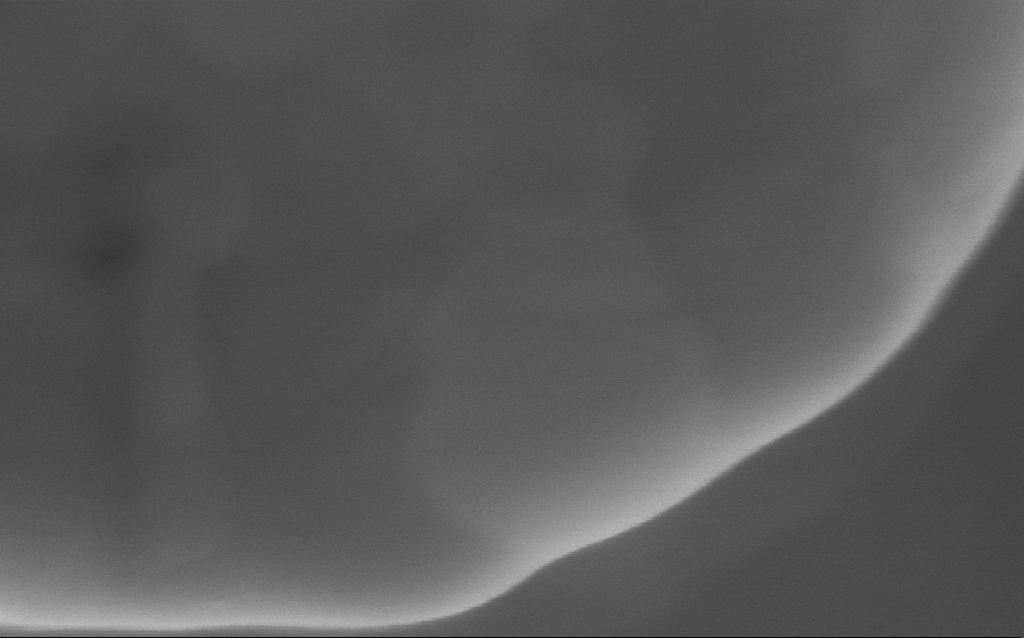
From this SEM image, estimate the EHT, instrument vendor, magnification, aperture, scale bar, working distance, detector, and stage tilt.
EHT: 5 kV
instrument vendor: Zeiss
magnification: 256 K X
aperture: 30 µm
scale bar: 200 nm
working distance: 4 mm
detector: InLens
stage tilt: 0°